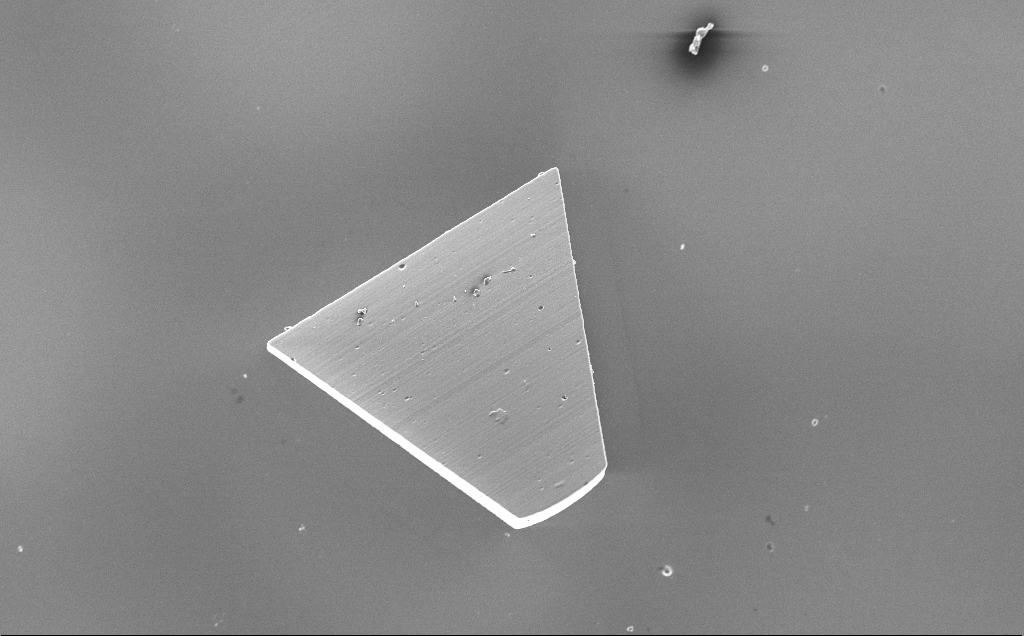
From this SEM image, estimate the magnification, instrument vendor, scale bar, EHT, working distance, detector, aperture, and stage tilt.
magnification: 0.314 K X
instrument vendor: Zeiss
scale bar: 100000 nm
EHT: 10 kV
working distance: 10 mm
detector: InLens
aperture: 30 µm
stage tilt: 0°